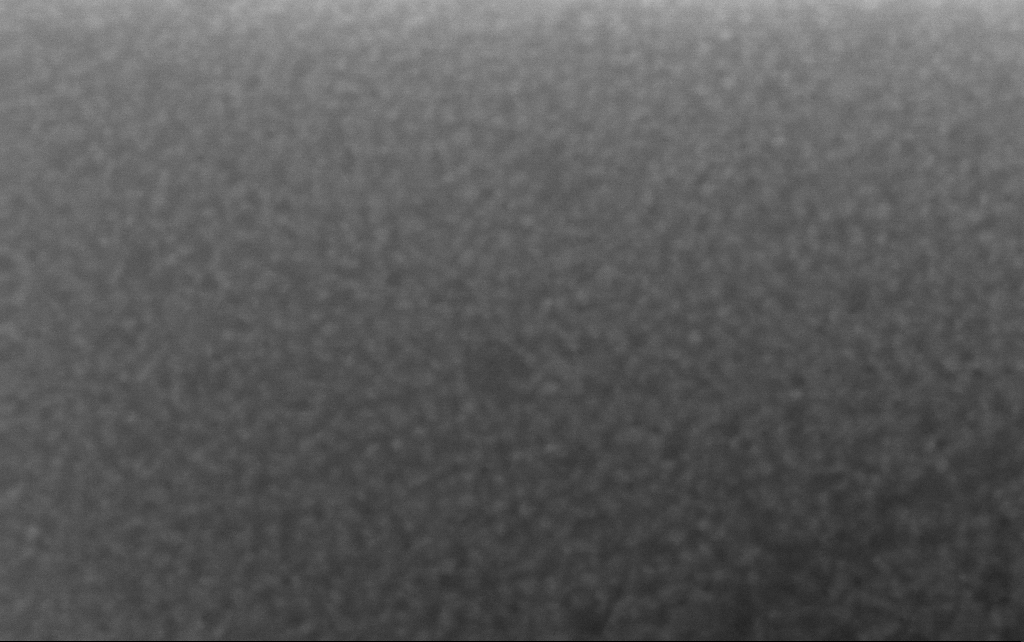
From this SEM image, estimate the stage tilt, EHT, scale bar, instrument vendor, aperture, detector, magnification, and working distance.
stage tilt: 0°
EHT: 10 kV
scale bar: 100 nm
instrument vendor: Zeiss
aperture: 30 µm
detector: InLens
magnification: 499.72 K X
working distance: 2.5 mm